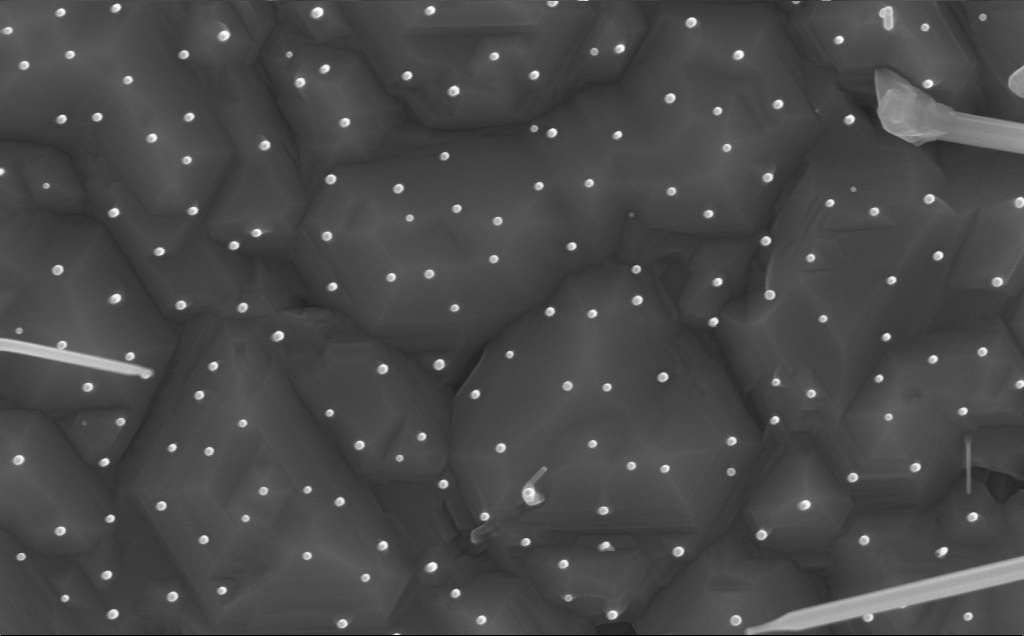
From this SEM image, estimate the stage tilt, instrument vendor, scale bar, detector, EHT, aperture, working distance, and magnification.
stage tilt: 0.2°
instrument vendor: Zeiss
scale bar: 200 nm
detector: InLens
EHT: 10 kV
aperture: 30 µm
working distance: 7 mm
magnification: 80 K X